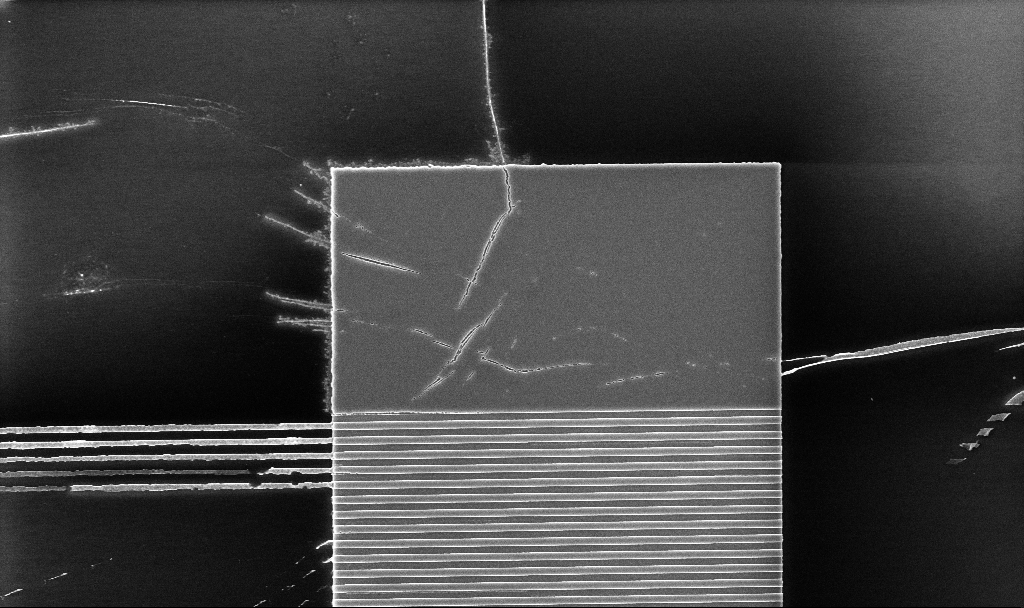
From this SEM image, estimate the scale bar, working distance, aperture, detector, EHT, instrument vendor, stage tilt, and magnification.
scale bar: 2000 nm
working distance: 5.2 mm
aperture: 30 µm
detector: InLens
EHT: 5 kV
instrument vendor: Zeiss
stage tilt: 0°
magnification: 8.33 K X